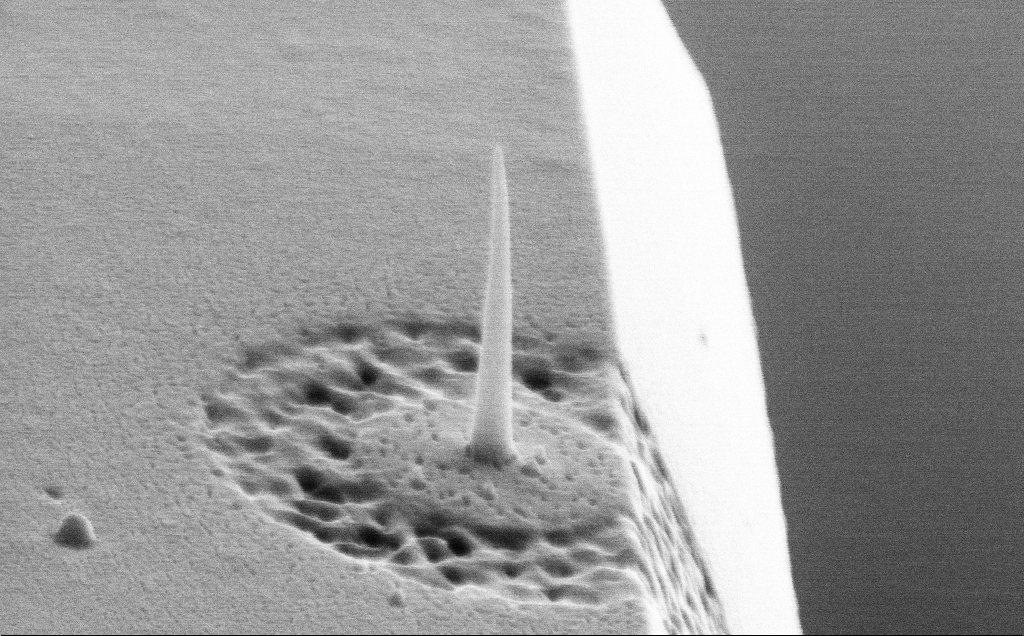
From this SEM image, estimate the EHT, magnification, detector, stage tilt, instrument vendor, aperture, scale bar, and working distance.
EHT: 10 kV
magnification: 53.36 K X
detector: SE2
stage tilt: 65.6°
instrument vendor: Zeiss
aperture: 30 µm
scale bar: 1000 nm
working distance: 10 mm